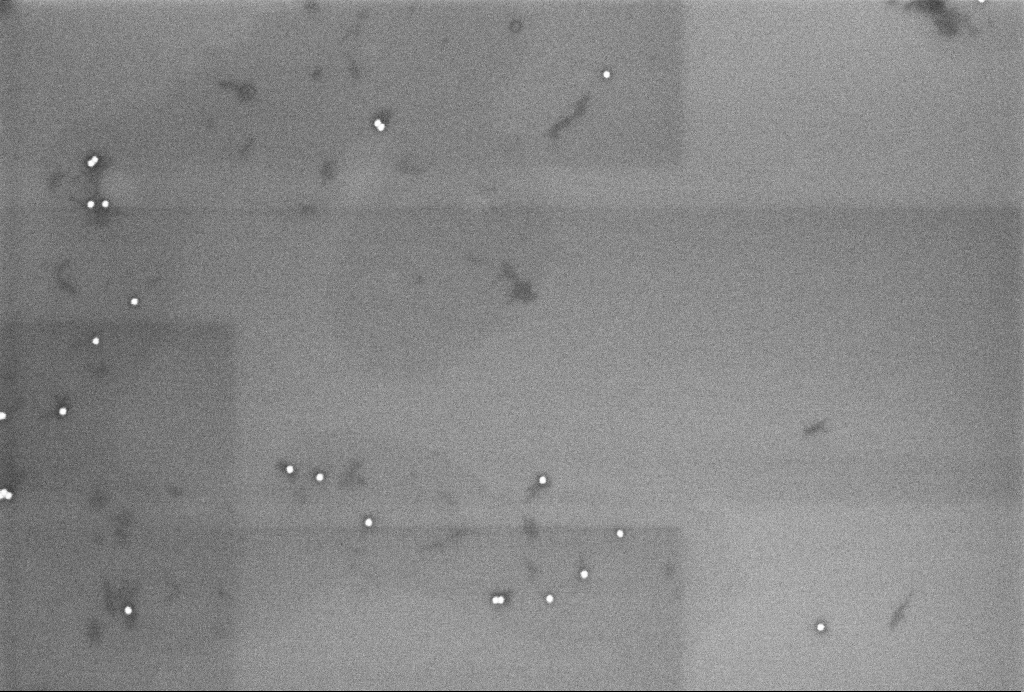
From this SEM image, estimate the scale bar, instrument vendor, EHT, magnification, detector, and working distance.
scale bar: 200 nm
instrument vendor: Zeiss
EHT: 2 kV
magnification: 83.83 K X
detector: InLens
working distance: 3.3 mm